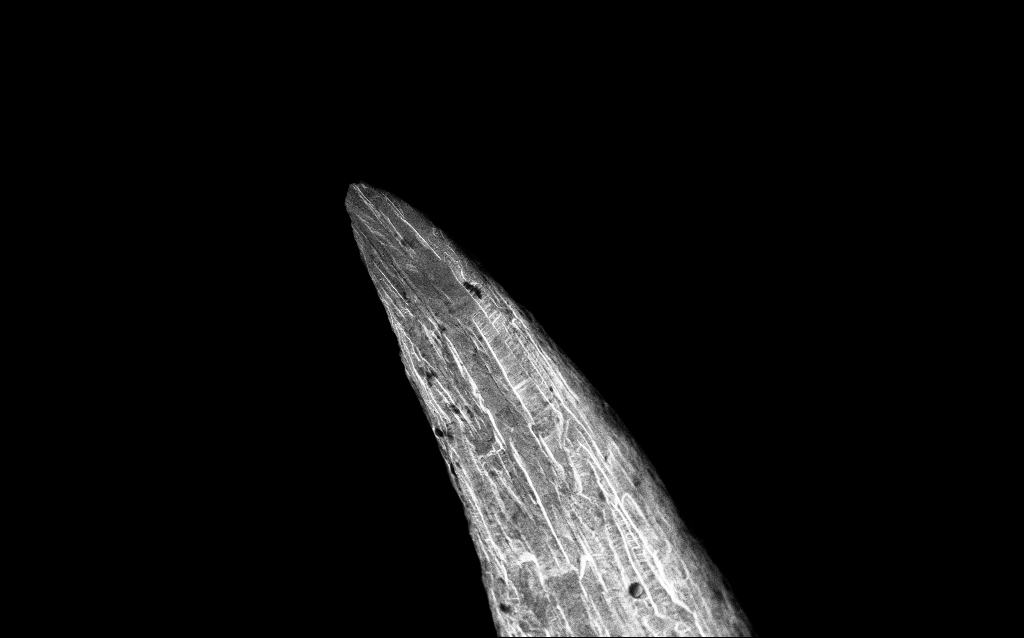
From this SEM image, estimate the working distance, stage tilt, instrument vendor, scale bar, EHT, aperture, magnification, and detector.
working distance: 4.3 mm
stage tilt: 45°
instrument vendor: Zeiss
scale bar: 10000 nm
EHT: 10 kV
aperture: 30 µm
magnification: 6.03 K X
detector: InLens